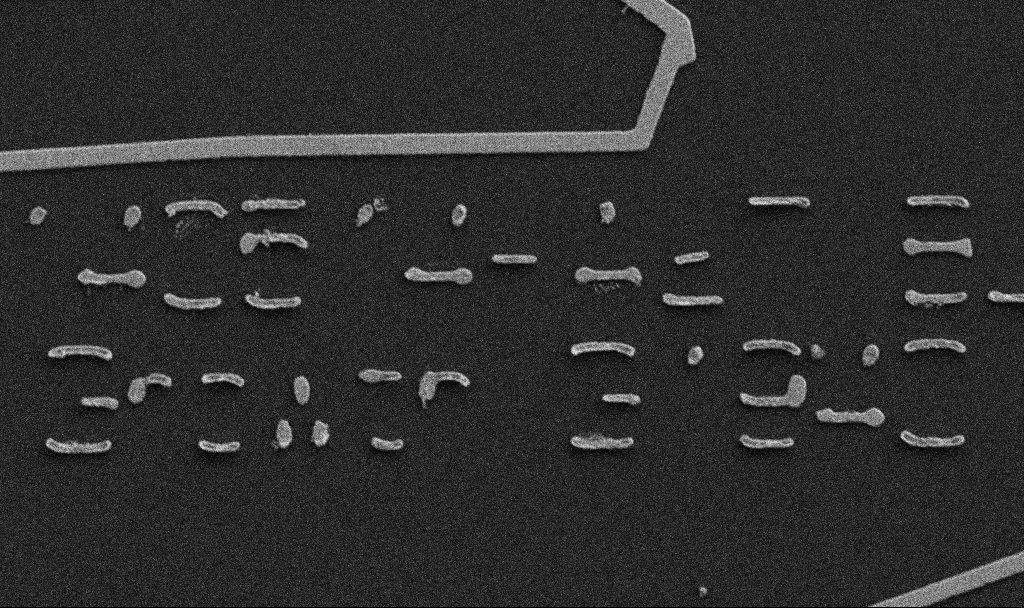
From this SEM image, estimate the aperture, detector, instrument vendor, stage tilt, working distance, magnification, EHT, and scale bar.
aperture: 30 µm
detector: SE2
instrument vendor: Zeiss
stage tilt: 0°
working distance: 10.7 mm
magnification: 9.85 K X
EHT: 5 kV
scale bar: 2000 nm